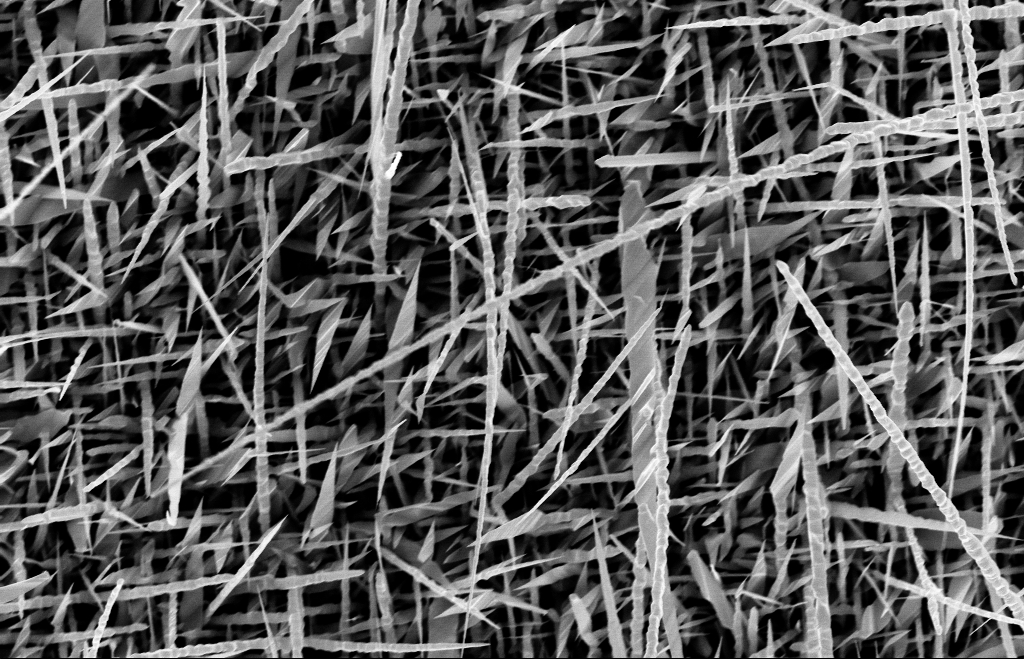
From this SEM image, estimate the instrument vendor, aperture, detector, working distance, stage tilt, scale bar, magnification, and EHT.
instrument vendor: Zeiss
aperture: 30 µm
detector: InLens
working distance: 8 mm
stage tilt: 0°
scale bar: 1000 nm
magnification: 20 K X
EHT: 10 kV